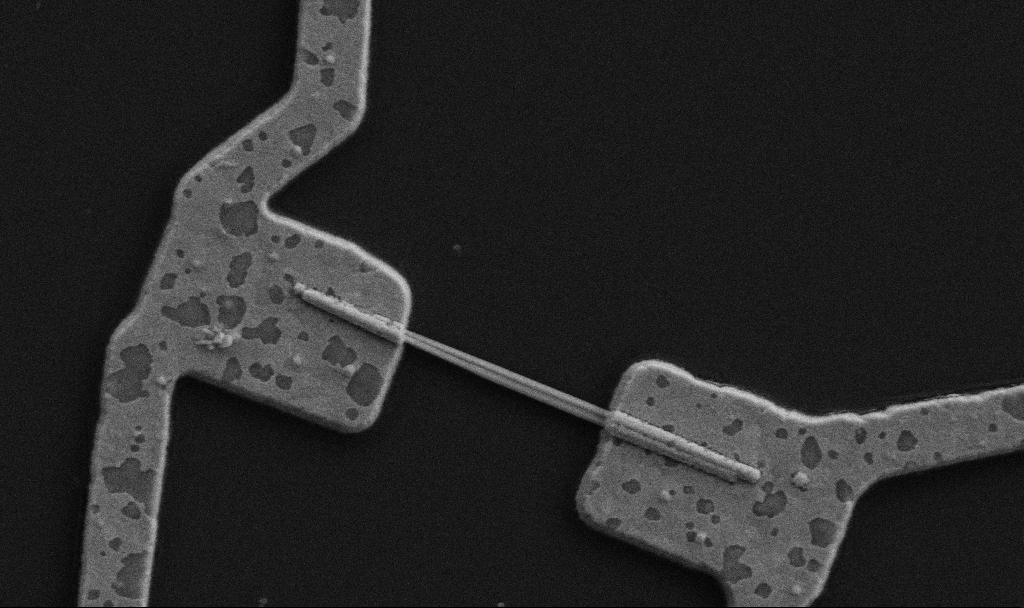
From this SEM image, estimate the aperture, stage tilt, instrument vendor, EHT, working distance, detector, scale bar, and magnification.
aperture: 30 µm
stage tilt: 0°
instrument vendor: Zeiss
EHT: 5 kV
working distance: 8.7 mm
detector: SE2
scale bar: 1000 nm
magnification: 30 K X